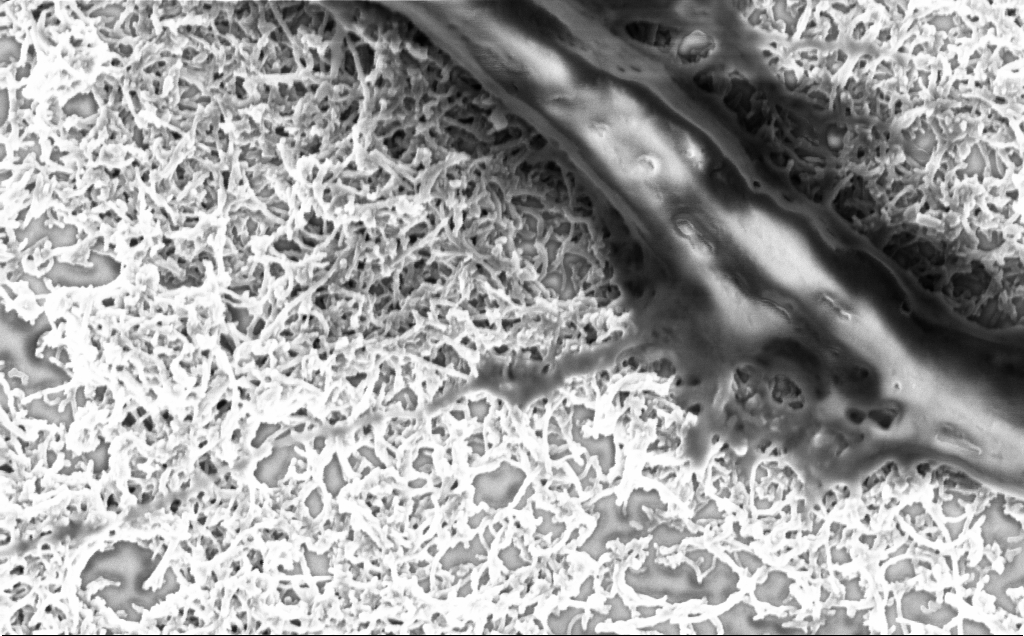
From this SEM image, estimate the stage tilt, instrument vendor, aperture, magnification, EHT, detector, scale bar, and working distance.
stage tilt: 0°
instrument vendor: Zeiss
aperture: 30 µm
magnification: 50 K X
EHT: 2 kV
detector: InLens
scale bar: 1000 nm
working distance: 7.1 mm